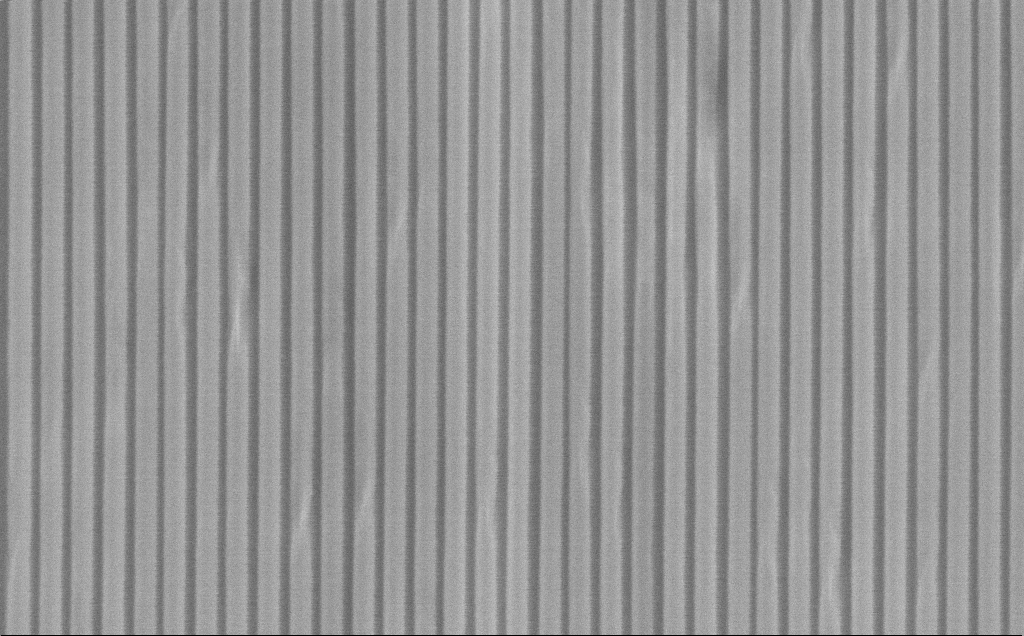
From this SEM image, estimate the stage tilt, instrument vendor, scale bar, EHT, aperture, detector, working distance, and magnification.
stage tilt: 45°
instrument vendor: Zeiss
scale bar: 1000 nm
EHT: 10 kV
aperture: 30 µm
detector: SE2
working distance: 10 mm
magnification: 64.23 K X